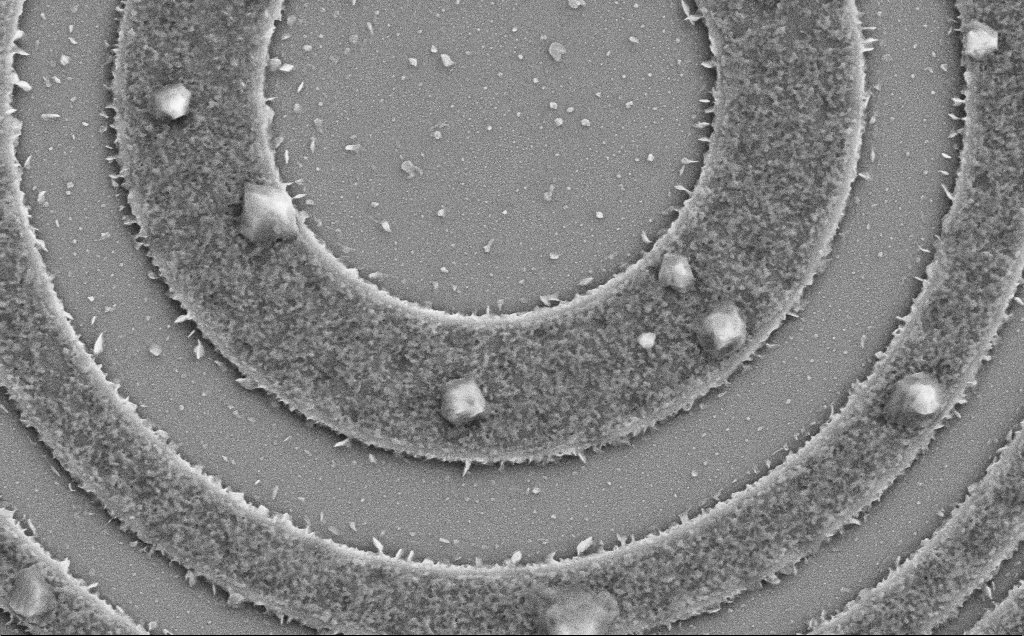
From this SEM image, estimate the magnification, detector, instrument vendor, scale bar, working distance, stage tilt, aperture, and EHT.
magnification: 22.57 K X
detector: SE2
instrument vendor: Zeiss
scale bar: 2000 nm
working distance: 6 mm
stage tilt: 0°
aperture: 30 µm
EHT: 5 kV